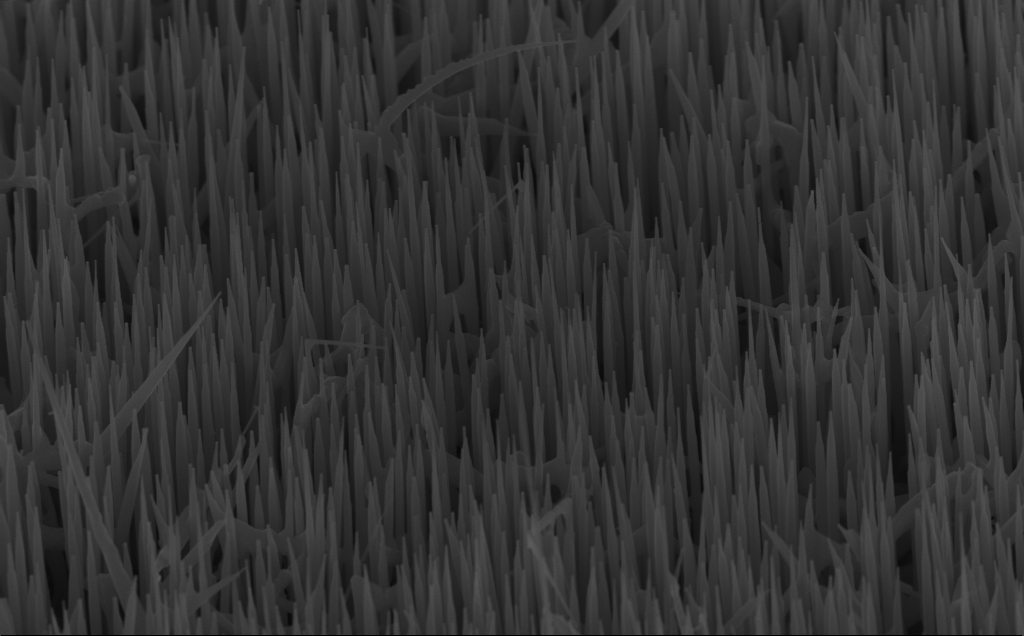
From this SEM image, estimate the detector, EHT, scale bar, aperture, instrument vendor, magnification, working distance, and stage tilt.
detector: InLens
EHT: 10 kV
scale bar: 1000 nm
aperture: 30 µm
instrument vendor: Zeiss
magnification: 40 K X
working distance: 6 mm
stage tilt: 45°